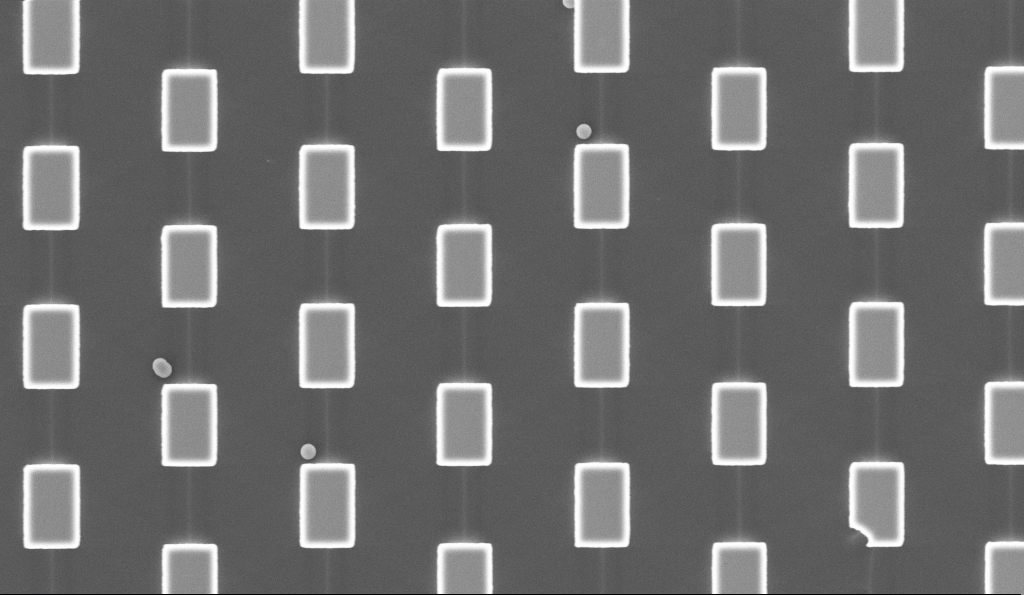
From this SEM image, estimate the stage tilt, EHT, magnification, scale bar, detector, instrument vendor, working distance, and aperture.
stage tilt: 0°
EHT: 5 kV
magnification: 9.71 K X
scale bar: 2000 nm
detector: InLens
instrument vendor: Zeiss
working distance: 3.1 mm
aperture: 30 µm